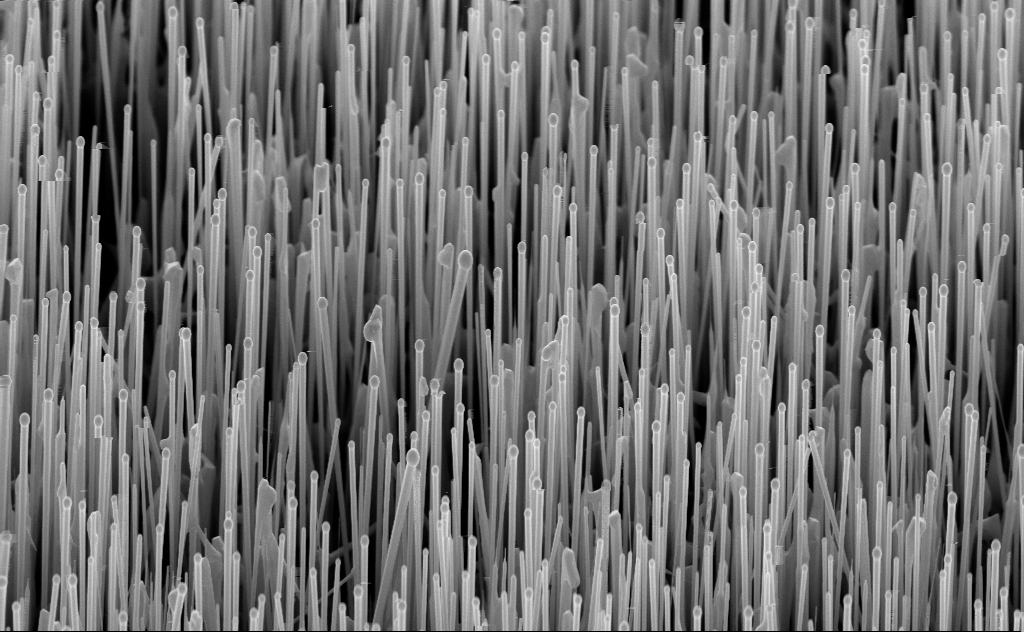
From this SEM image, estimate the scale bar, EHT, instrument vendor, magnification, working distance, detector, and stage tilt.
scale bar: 2000 nm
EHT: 10 kV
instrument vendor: Zeiss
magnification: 20 K X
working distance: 6 mm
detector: InLens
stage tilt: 45°